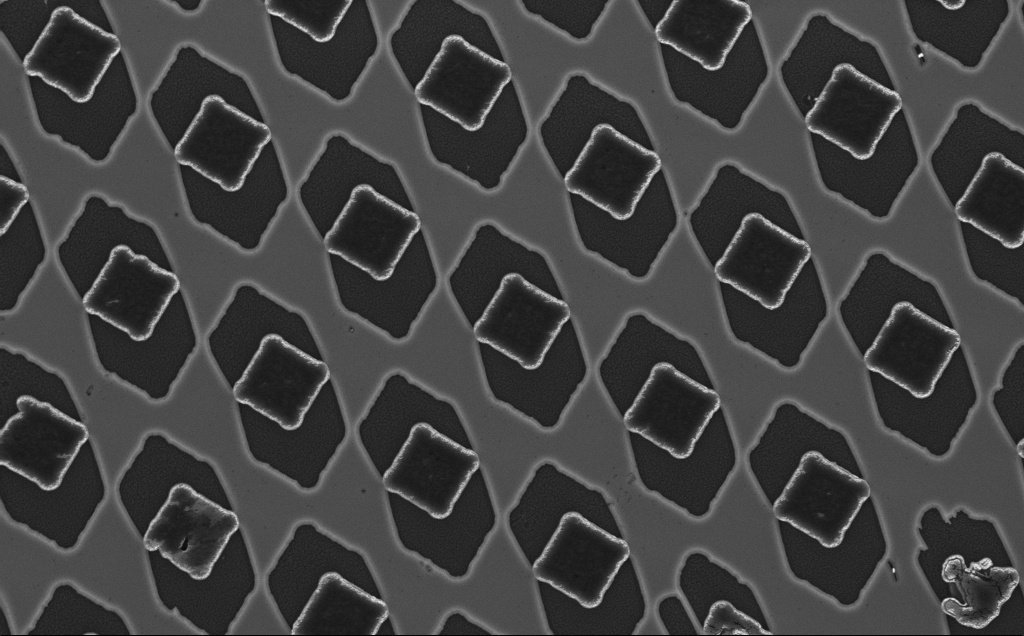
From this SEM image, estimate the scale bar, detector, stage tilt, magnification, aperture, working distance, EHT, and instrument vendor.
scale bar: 10000 nm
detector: InLens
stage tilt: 0°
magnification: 3.22 K X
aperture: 30 µm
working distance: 9 mm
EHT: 10 kV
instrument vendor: Zeiss